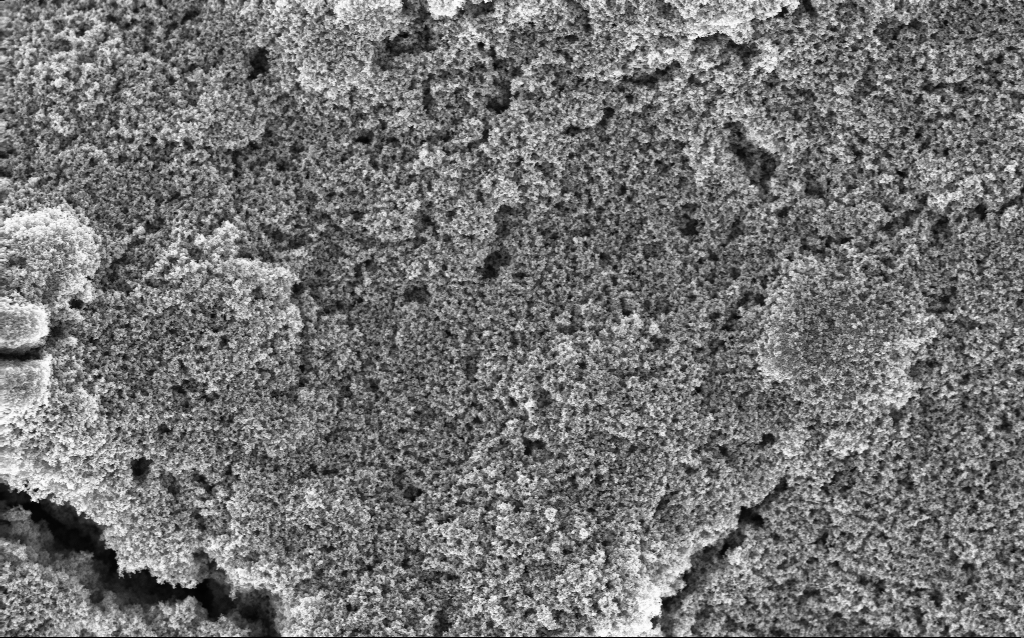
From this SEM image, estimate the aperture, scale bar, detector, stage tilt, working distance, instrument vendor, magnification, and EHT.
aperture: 30 µm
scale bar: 1000 nm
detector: InLens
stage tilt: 0°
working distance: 2.9 mm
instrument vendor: Zeiss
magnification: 23.9 K X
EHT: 5 kV